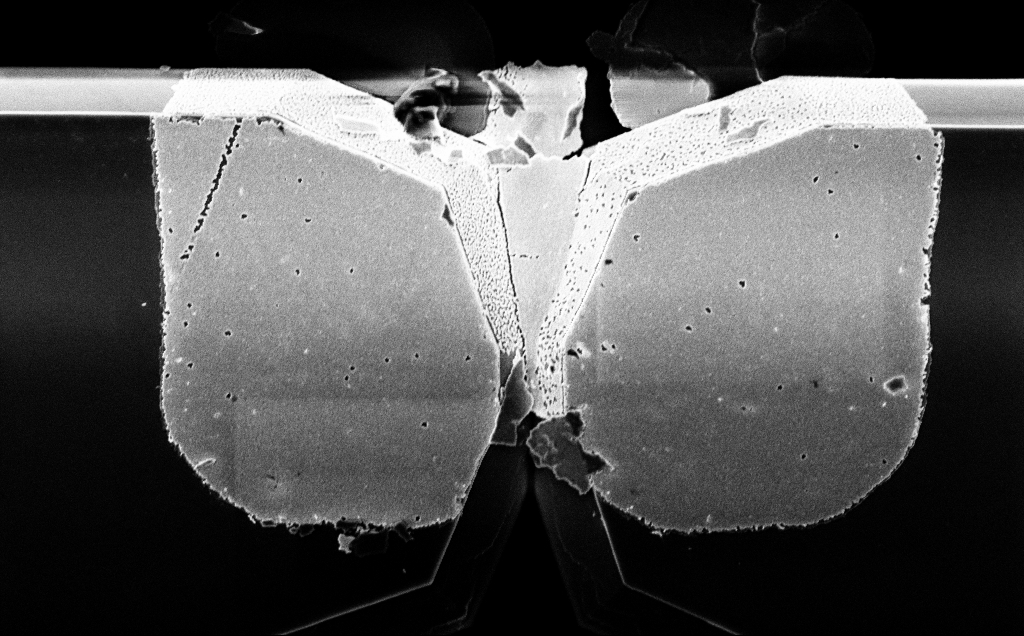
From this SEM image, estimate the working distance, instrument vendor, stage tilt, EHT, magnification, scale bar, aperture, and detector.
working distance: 15 mm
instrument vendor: Zeiss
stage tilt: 0.2°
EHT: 5 kV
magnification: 10.7 K X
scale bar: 2000 nm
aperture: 30 µm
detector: InLens